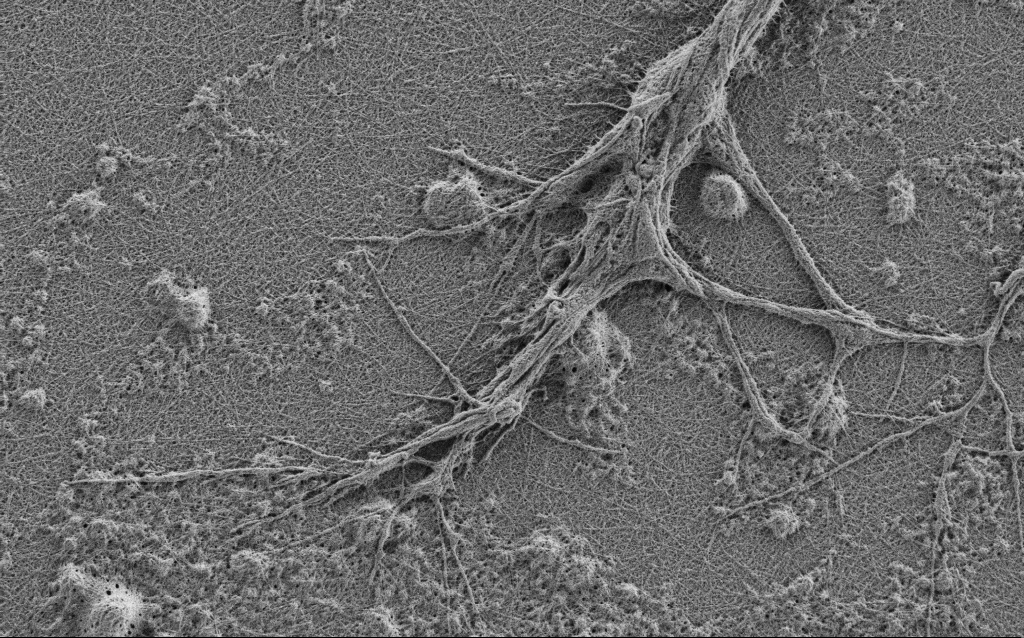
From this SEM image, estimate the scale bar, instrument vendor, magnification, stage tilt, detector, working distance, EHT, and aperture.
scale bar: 2000 nm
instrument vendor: Zeiss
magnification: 10 K X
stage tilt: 0°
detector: SE2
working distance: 4 mm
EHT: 0.9 kV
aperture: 30 µm